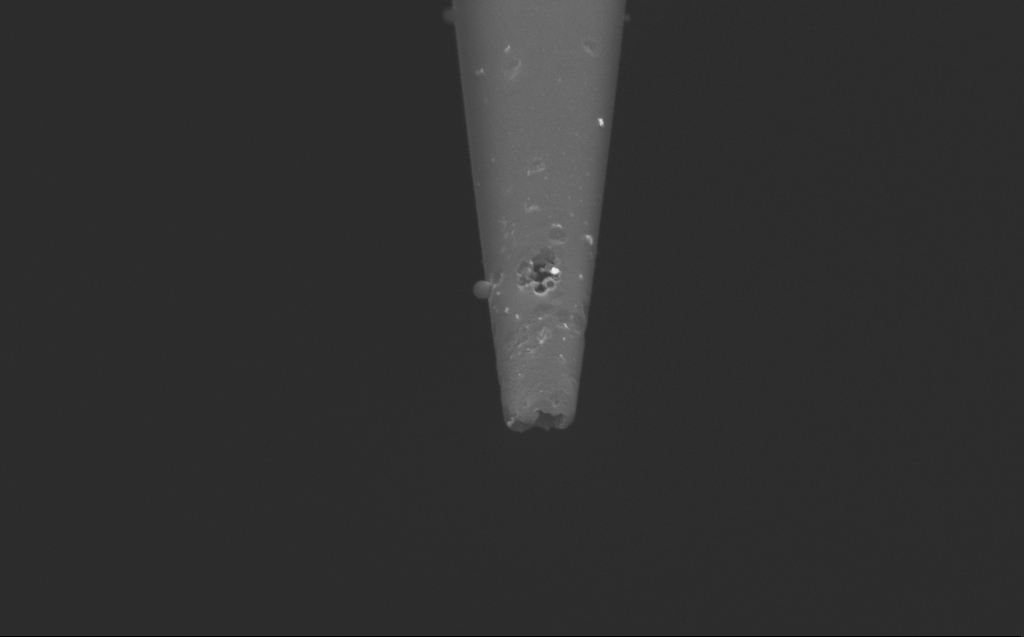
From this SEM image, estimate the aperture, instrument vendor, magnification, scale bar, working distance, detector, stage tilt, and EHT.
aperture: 30 µm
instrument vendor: Zeiss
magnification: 58.93 K X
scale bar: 1000 nm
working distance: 4 mm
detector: InLens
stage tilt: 45°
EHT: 5 kV